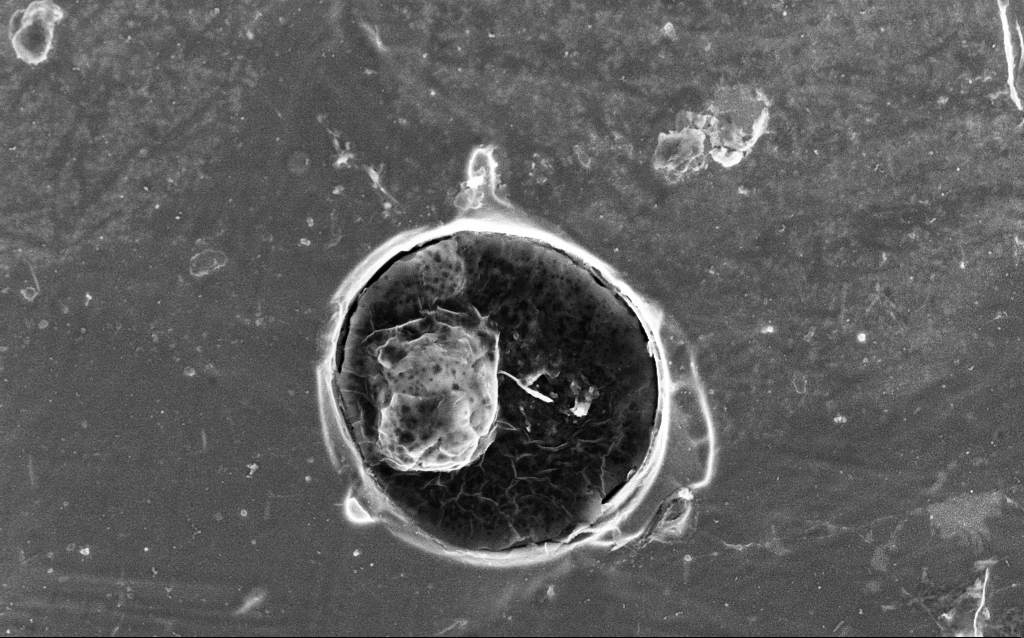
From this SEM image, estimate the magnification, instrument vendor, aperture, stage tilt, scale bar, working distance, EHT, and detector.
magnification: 22.29 K X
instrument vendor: Zeiss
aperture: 30 µm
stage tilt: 0°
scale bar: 1000 nm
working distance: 5.5 mm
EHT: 5 kV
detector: InLens